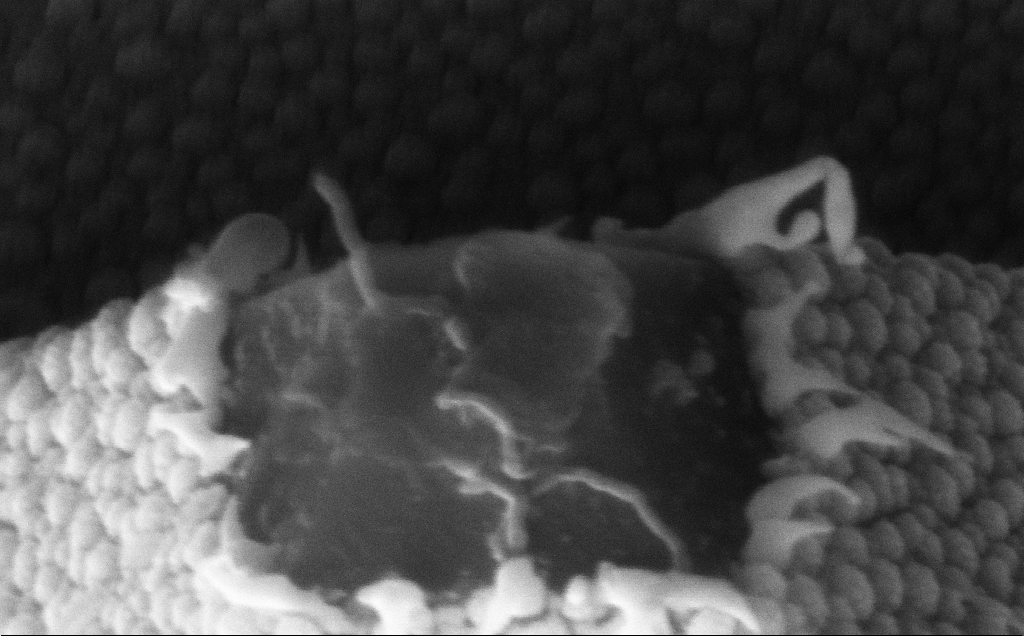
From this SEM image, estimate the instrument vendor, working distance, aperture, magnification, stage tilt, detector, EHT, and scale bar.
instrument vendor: Zeiss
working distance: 11 mm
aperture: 30 µm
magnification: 232.91 K X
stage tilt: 45°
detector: InLens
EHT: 10 kV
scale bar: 200 nm